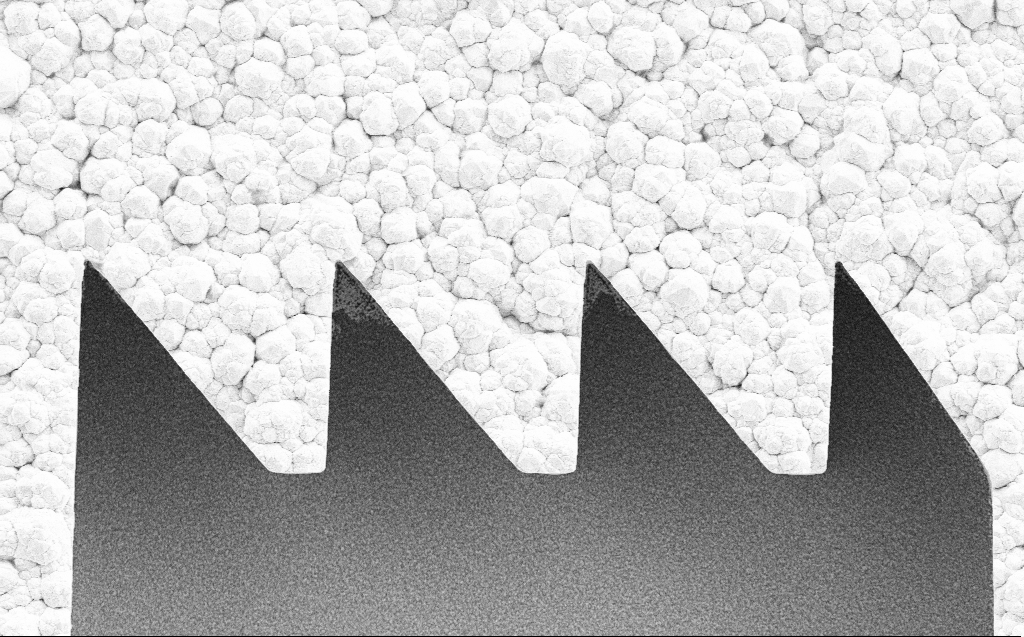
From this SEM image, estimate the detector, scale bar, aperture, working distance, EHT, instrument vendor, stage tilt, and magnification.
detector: SE2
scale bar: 2000 nm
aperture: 30 µm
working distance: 6 mm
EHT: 5 kV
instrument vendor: Zeiss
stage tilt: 0°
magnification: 8.1 K X